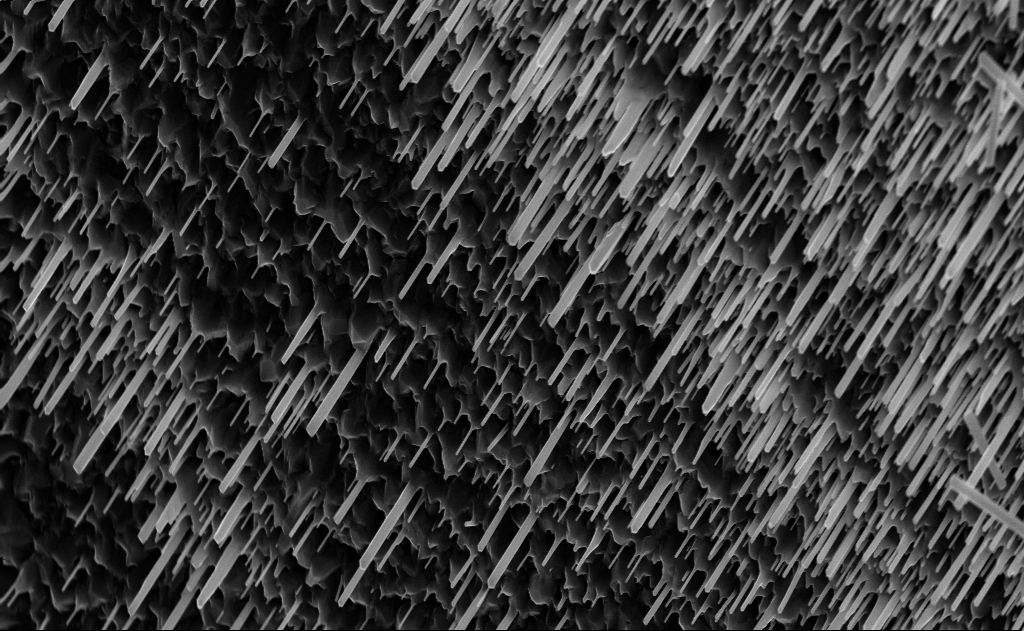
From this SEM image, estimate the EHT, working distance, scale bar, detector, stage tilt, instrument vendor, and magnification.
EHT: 10 kV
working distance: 7 mm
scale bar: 2000 nm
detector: InLens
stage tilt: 0°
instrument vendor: Zeiss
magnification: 20 K X